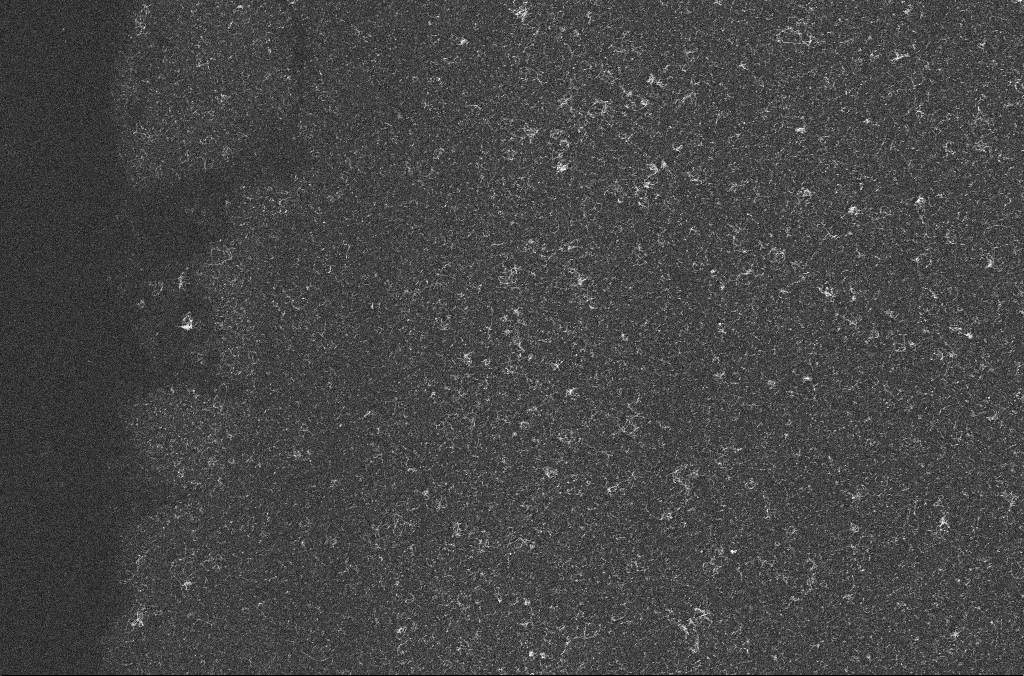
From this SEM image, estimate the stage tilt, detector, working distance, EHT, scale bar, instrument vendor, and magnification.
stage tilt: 0°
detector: InLens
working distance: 3.3 mm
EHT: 10 kV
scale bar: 2000 nm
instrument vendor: Zeiss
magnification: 10 K X